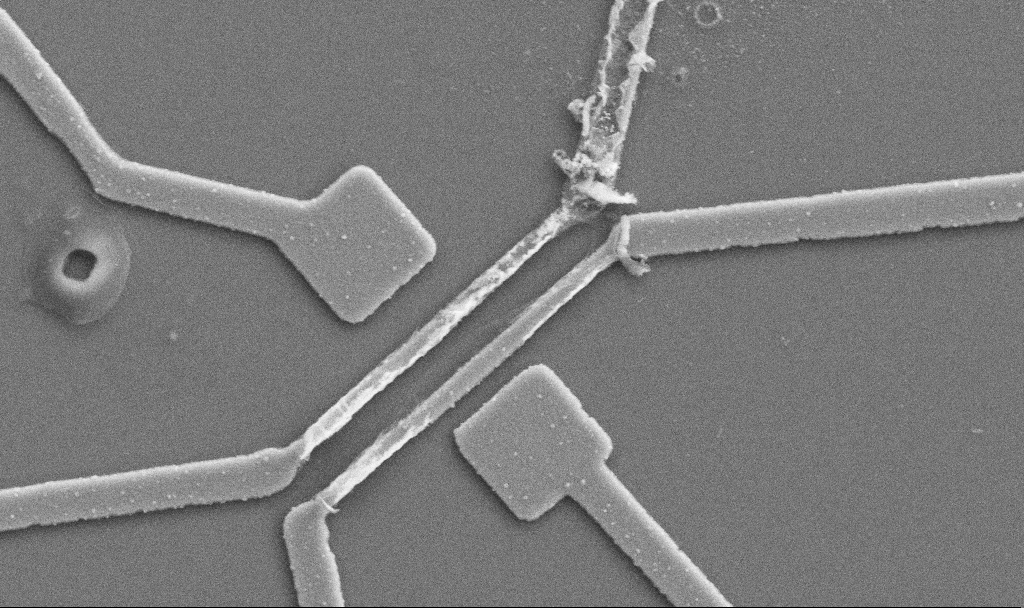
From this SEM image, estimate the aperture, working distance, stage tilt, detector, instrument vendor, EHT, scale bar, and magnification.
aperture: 30 µm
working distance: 10.7 mm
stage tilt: -0°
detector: SE2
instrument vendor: Zeiss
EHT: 5 kV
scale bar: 1000 nm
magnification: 20 K X